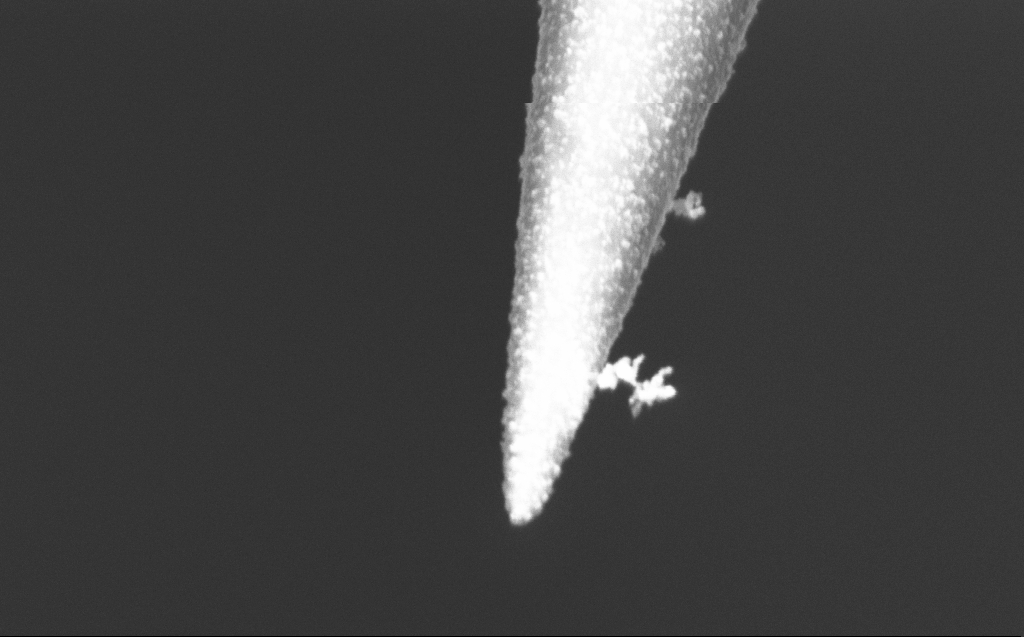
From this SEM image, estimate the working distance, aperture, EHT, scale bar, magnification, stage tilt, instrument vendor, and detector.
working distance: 6 mm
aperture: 30 µm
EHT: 2 kV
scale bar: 200 nm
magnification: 80 K X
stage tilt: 45°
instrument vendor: Zeiss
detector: InLens